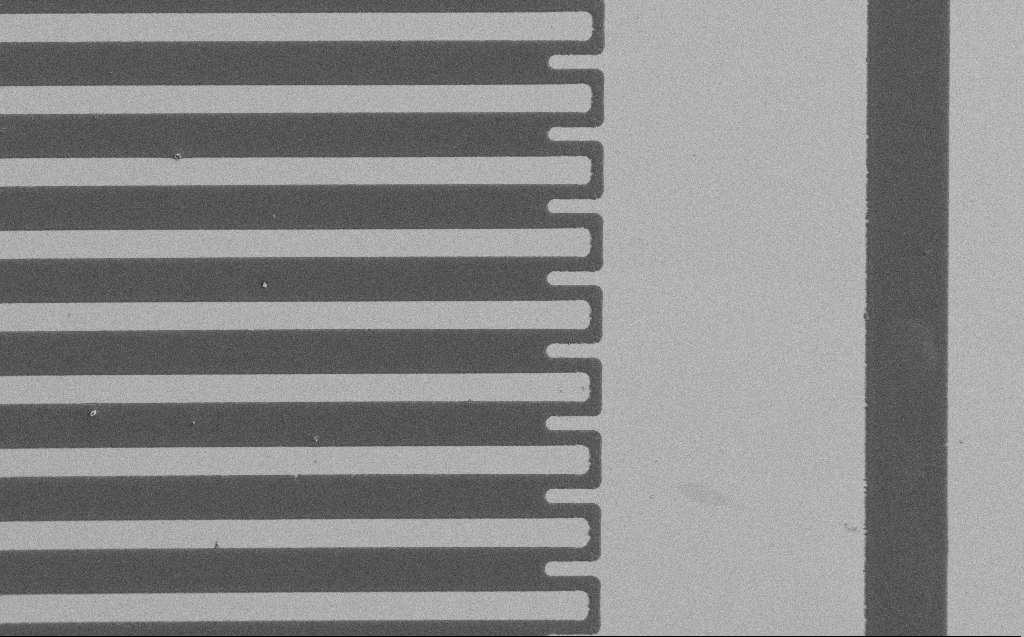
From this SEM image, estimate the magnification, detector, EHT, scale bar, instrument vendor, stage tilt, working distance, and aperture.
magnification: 0.968 K X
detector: SE2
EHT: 1.2 kV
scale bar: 20000 nm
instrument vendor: Zeiss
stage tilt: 0°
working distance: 6 mm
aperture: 30 µm